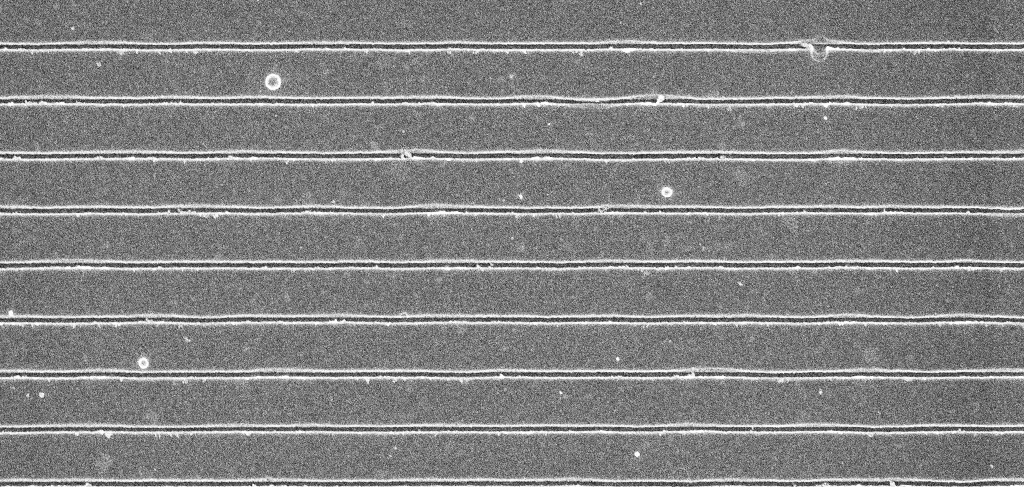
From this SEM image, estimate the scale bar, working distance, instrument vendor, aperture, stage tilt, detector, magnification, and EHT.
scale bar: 2000 nm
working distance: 5.3 mm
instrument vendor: Zeiss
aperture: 30 µm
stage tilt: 0°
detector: InLens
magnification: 22.46 K X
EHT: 5 kV